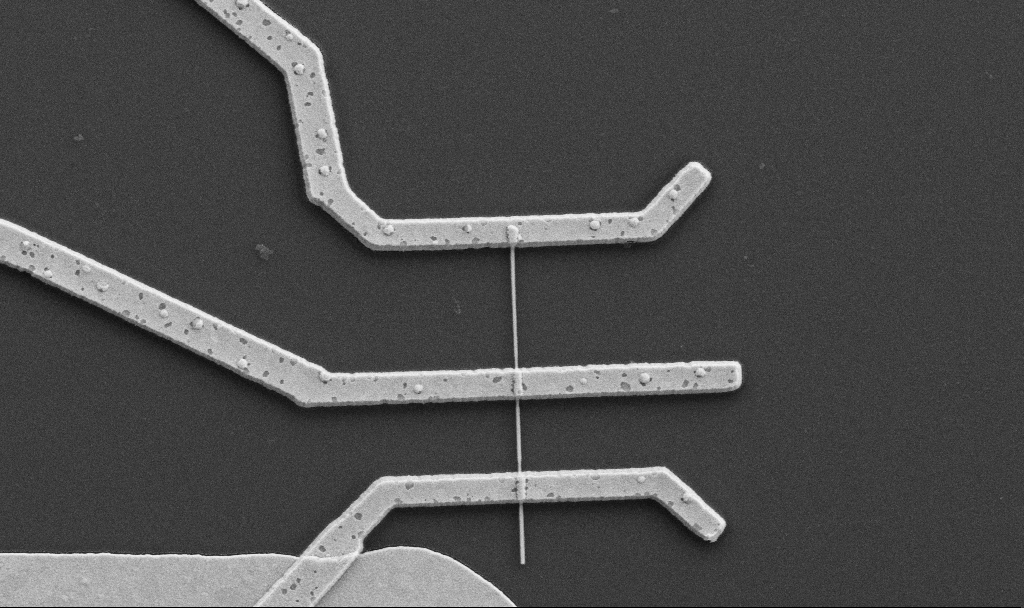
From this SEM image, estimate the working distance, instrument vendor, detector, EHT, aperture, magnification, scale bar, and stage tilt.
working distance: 10.6 mm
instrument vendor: Zeiss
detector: SE2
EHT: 5 kV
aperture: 30 µm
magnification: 20 K X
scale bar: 1000 nm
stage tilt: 0°